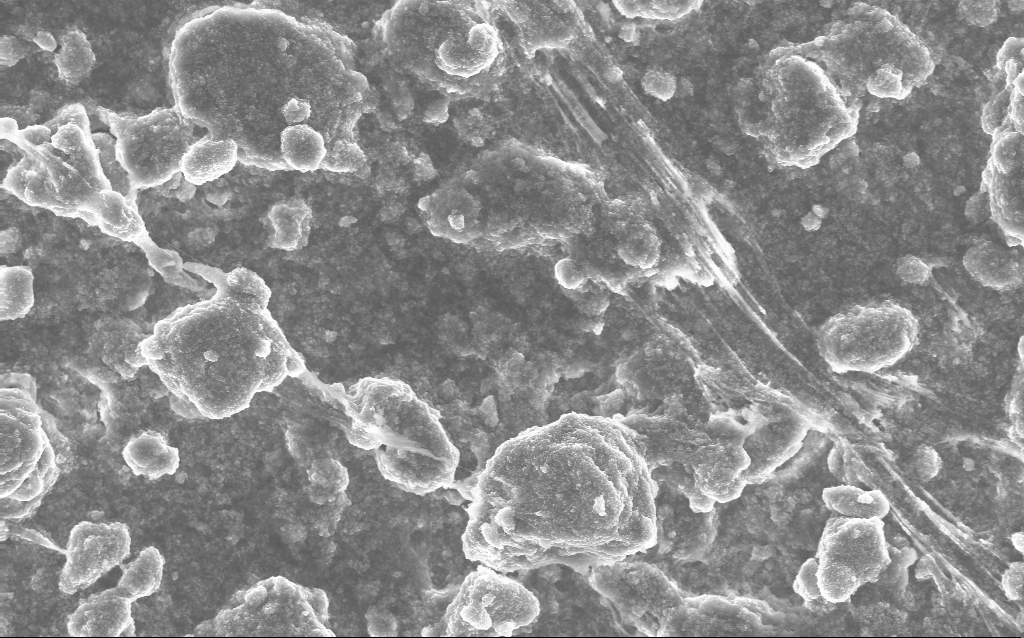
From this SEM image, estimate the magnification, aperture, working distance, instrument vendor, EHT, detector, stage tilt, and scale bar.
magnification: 5.83 K X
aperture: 30 µm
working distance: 2.7 mm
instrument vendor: Zeiss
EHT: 10 kV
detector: InLens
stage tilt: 0°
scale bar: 10000 nm